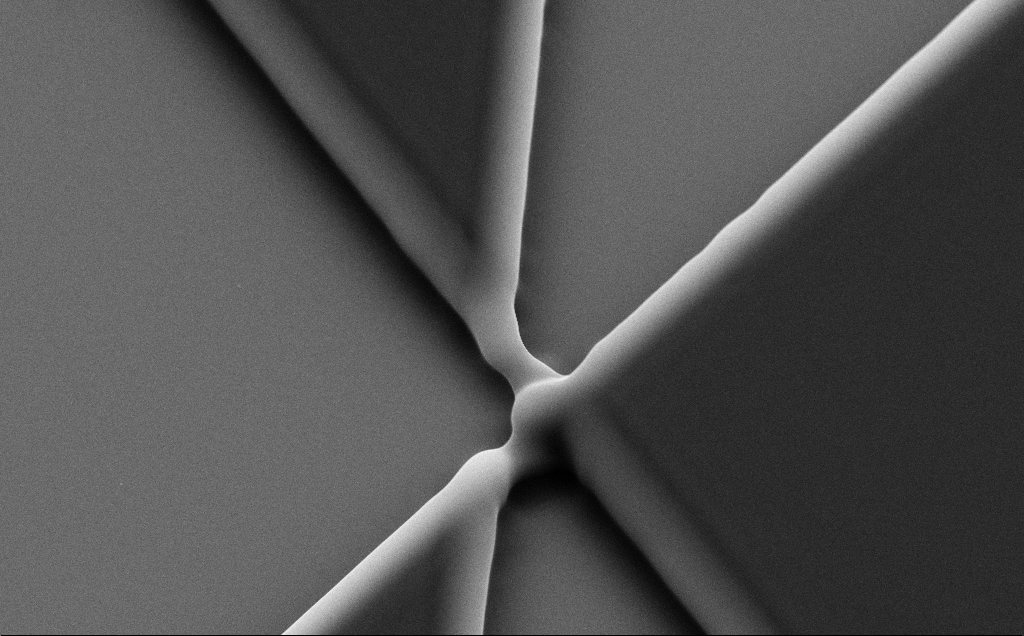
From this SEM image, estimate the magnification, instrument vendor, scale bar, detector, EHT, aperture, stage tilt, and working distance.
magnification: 11.83 K X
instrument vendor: Zeiss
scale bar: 2000 nm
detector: SE2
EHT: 10 kV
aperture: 30 µm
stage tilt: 35°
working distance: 8 mm